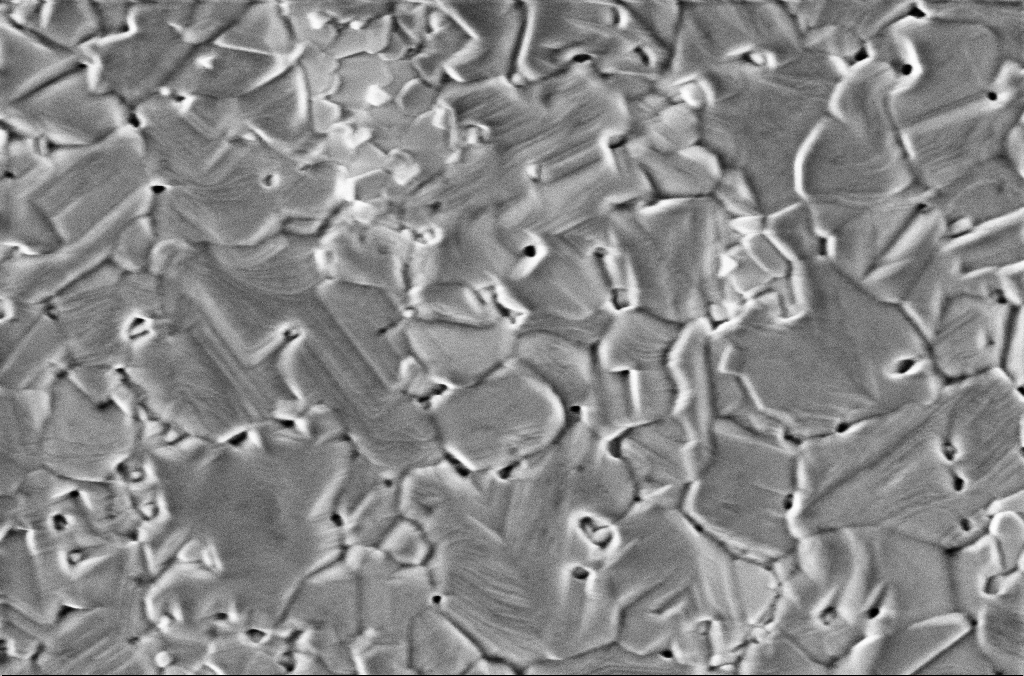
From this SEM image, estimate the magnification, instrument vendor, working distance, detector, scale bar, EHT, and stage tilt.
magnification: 70 K X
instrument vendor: Zeiss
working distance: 3 mm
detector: InLens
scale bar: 200 nm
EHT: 2 kV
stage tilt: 0°